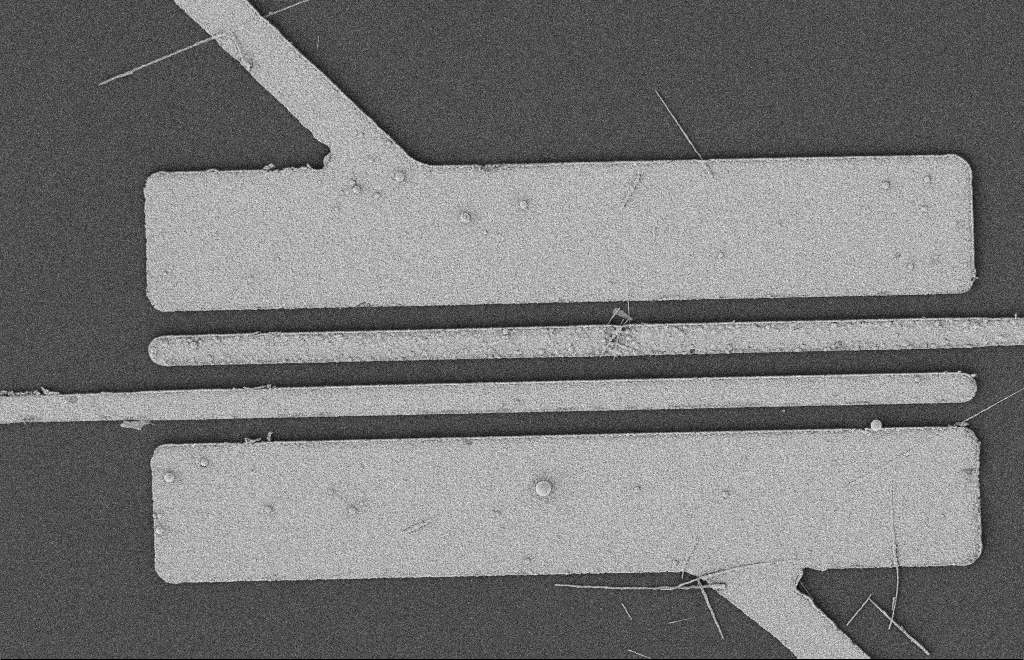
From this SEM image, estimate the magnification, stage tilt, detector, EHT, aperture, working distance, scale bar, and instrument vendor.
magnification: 4.96 K X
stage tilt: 0°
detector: SE2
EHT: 2 kV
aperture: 20 µm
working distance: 12 mm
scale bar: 2000 nm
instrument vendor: Zeiss